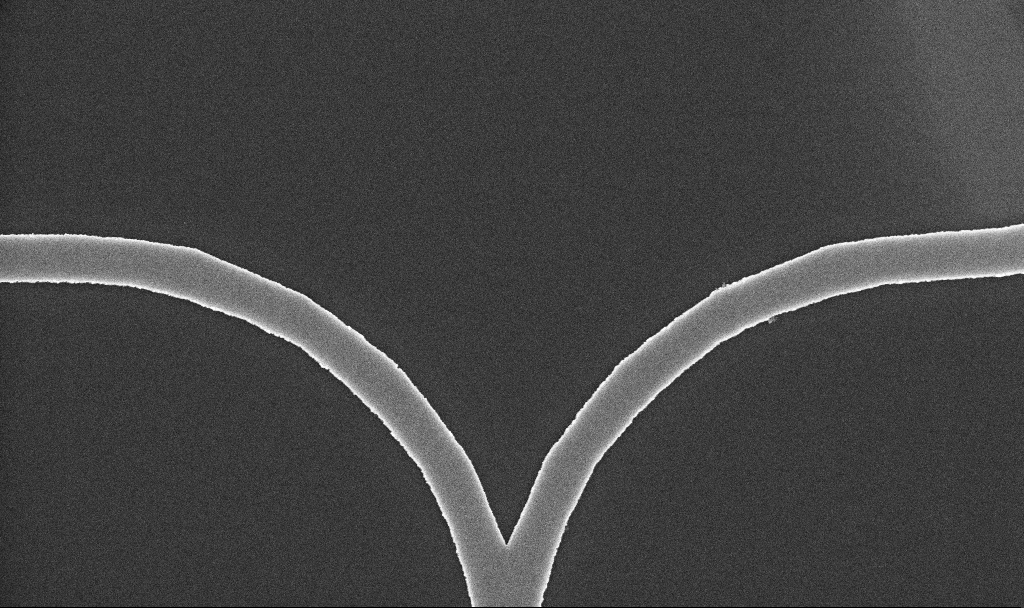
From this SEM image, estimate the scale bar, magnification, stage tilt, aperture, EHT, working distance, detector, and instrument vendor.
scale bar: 1000 nm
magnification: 33.47 K X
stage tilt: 0°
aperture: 30 µm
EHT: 5 kV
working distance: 10.3 mm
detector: InLens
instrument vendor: Zeiss